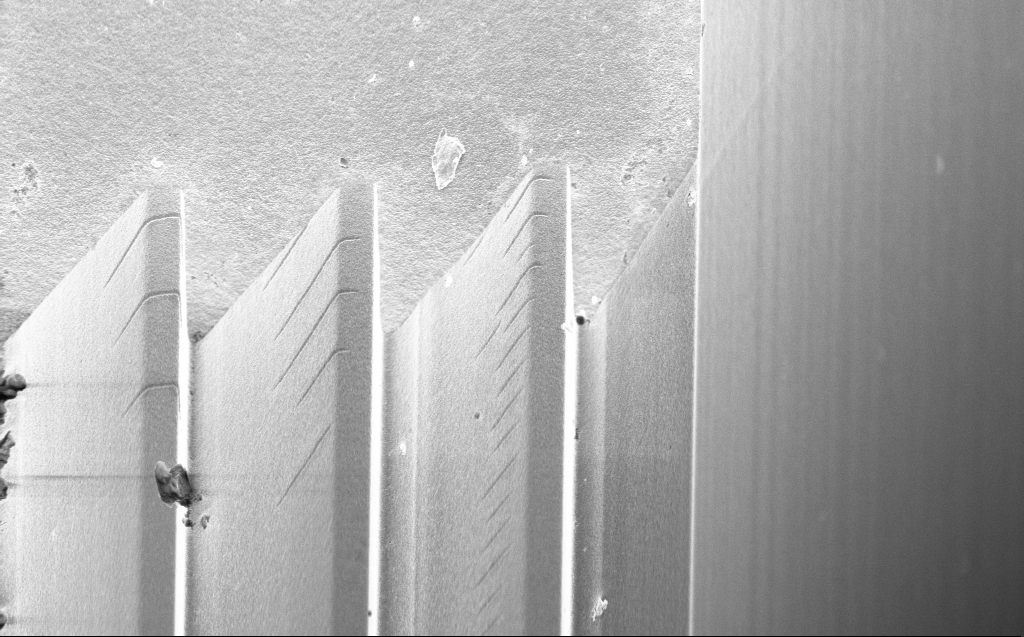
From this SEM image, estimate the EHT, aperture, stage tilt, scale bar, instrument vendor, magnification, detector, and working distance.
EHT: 3 kV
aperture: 30 µm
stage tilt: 45°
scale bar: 10000 nm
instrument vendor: Zeiss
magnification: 6.27 K X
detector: InLens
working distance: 5 mm